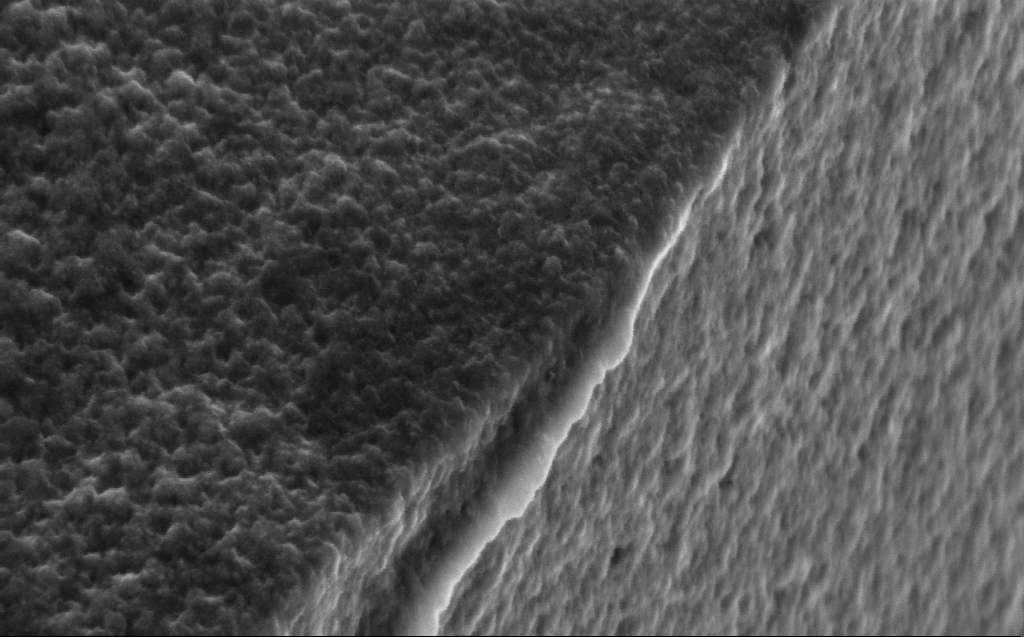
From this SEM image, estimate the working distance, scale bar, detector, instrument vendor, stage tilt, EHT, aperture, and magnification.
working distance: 5 mm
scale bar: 200 nm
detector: InLens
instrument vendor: Zeiss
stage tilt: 45°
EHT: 5 kV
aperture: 30 µm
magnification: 119.73 K X